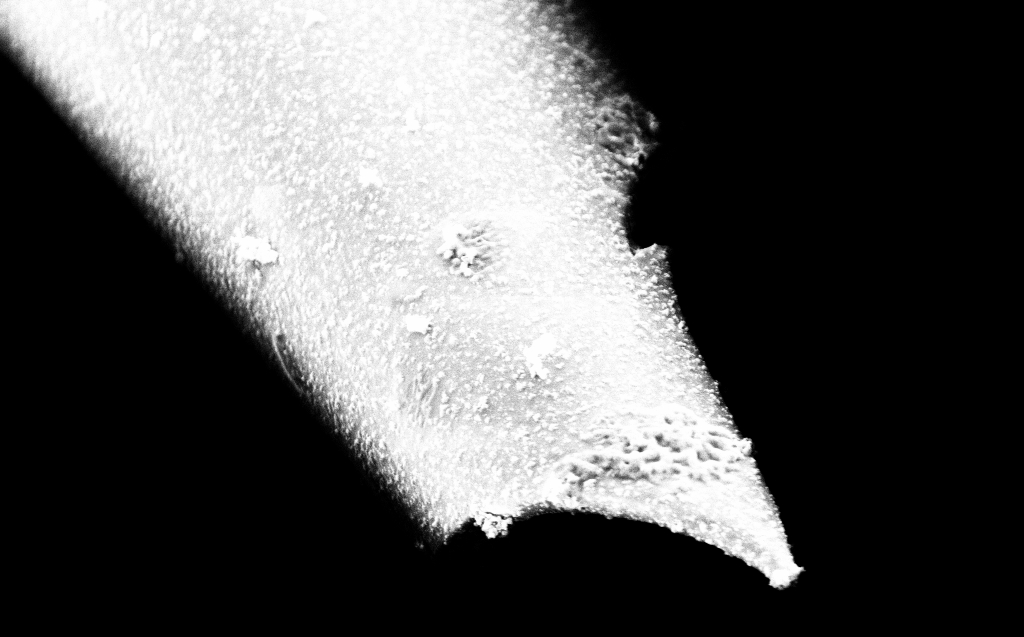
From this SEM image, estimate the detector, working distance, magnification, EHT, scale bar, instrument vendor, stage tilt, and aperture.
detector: InLens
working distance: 4 mm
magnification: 50 K X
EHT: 2 kV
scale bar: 1000 nm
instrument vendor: Zeiss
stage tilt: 45°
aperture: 30 µm